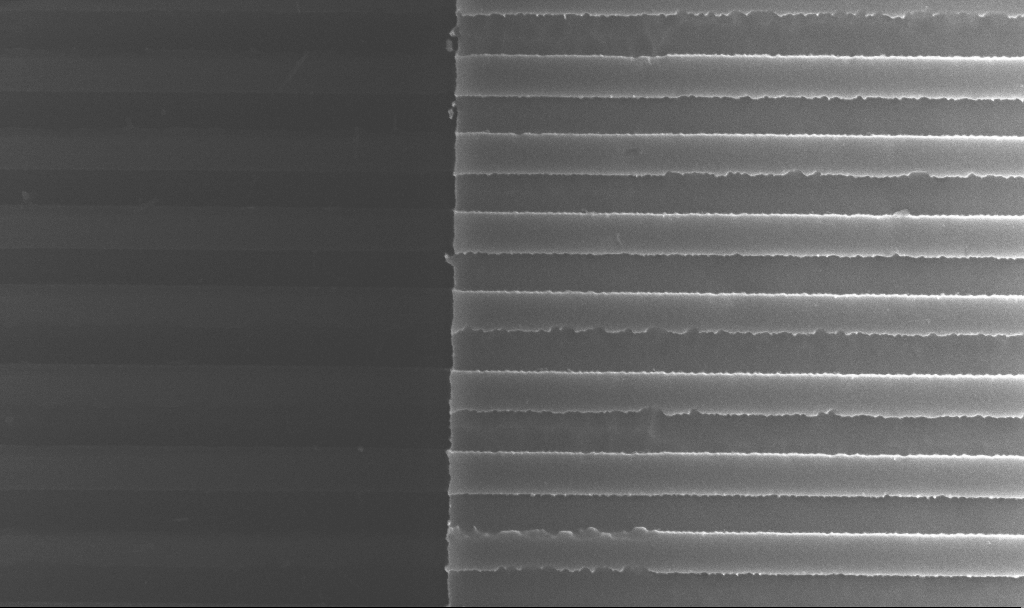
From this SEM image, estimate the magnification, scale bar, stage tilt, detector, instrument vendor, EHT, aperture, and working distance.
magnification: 44.61 K X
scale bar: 1000 nm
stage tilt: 0°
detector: InLens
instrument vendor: Zeiss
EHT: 5 kV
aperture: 30 µm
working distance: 10 mm